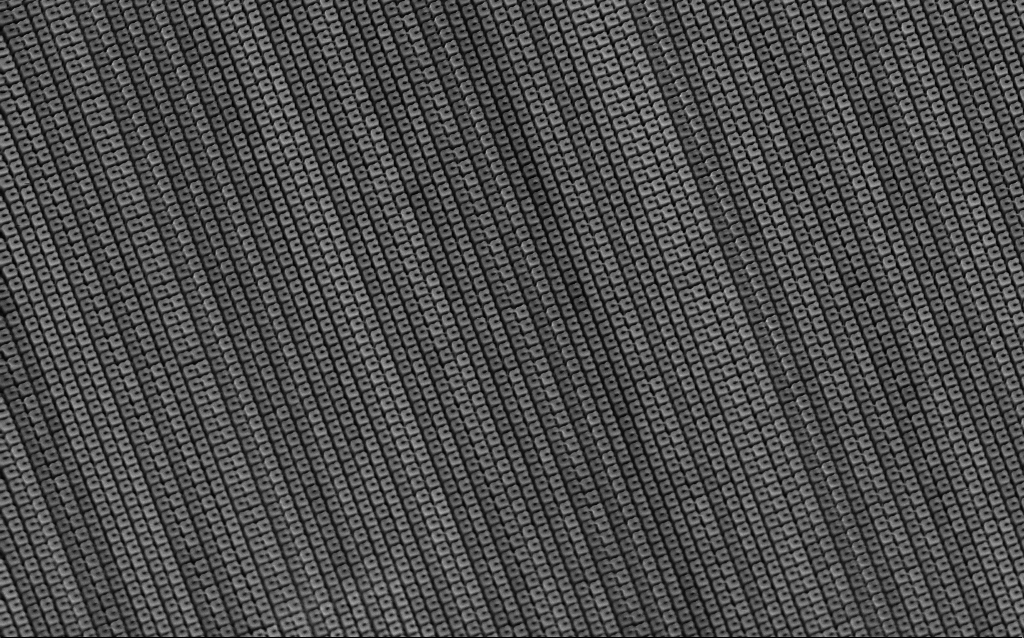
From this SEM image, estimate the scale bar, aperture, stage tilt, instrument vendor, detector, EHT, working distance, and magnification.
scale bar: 2000 nm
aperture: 30 µm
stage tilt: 30°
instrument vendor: Zeiss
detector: InLens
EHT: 1.5 kV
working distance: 6.4 mm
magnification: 11.34 K X